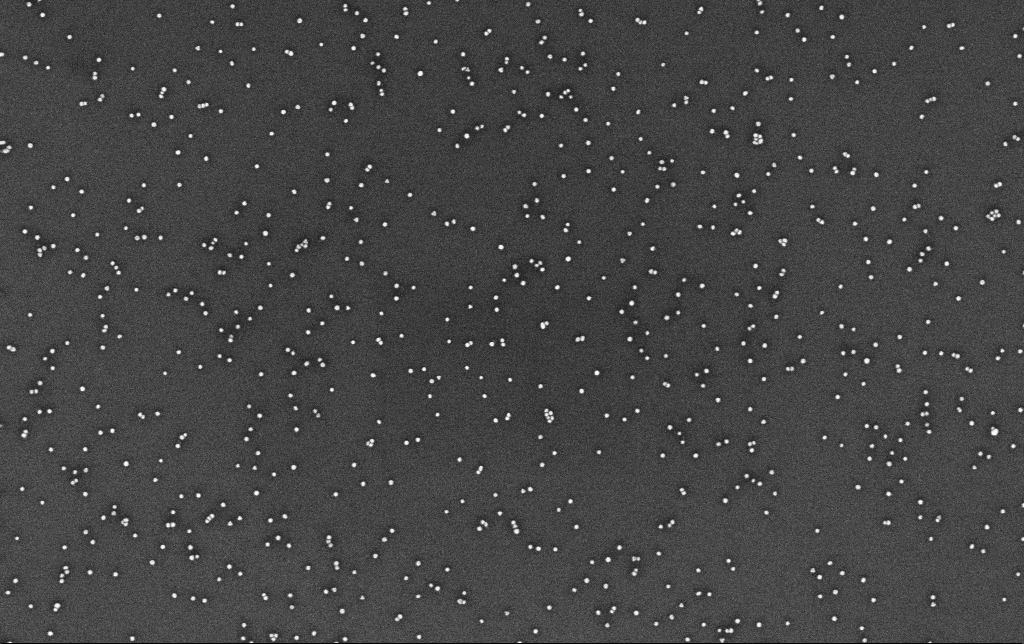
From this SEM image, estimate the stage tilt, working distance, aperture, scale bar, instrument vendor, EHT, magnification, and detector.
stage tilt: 0°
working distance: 3.3 mm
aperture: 30 µm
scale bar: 200 nm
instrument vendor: Zeiss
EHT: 10 kV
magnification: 100 K X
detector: InLens